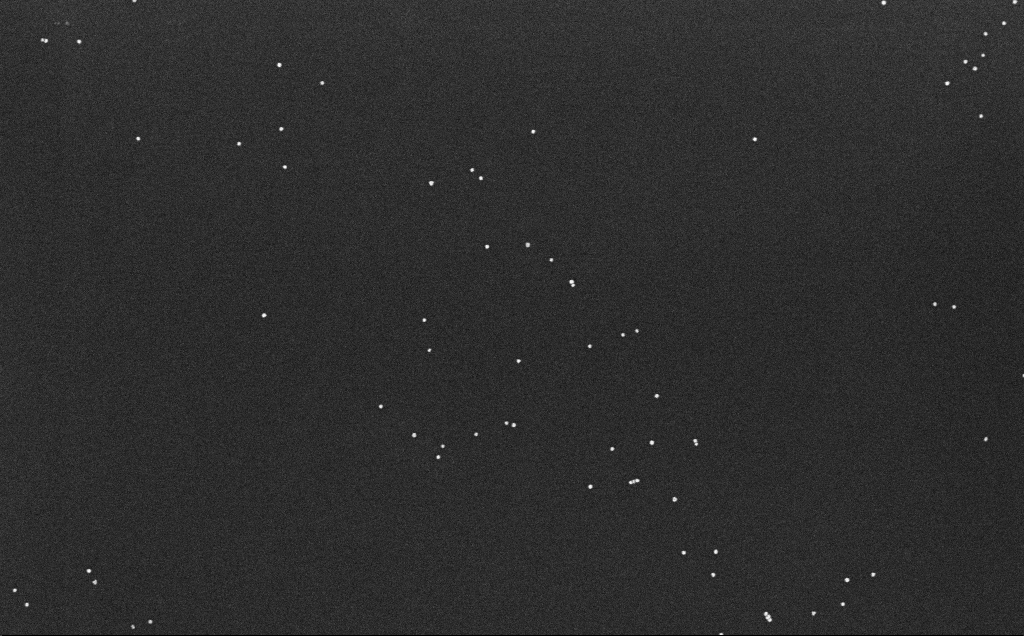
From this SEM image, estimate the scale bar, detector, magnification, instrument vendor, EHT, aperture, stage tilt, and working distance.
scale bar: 200 nm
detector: InLens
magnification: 100 K X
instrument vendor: Zeiss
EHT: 10 kV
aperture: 30 µm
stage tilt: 0°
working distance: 6.6 mm